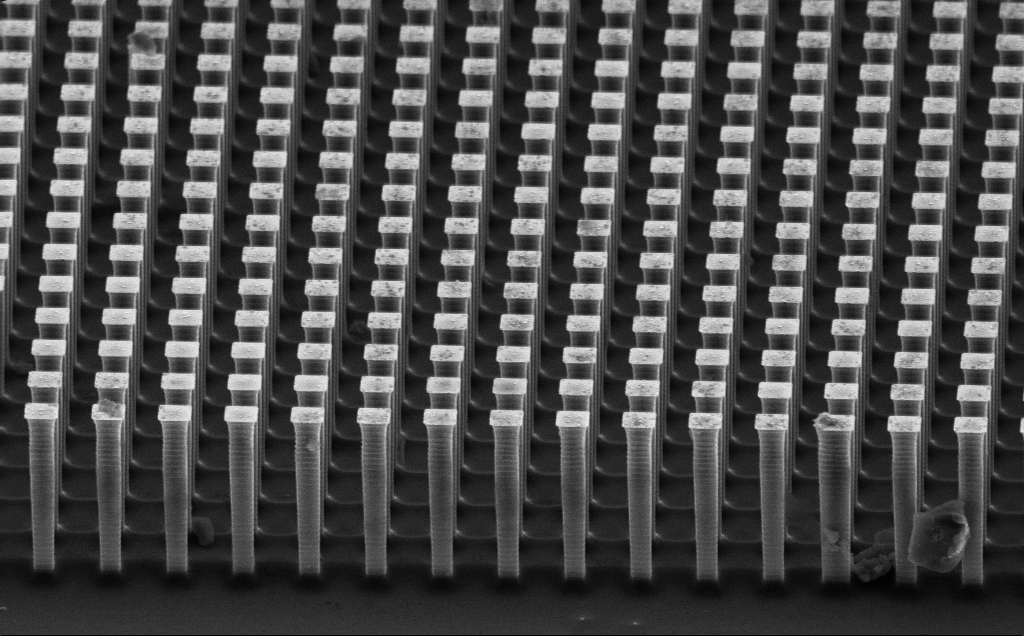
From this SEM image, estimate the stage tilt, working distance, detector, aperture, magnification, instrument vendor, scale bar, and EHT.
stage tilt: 60.5°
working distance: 15 mm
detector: SE2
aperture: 30 µm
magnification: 6.1 K X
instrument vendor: Zeiss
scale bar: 10000 nm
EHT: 10 kV